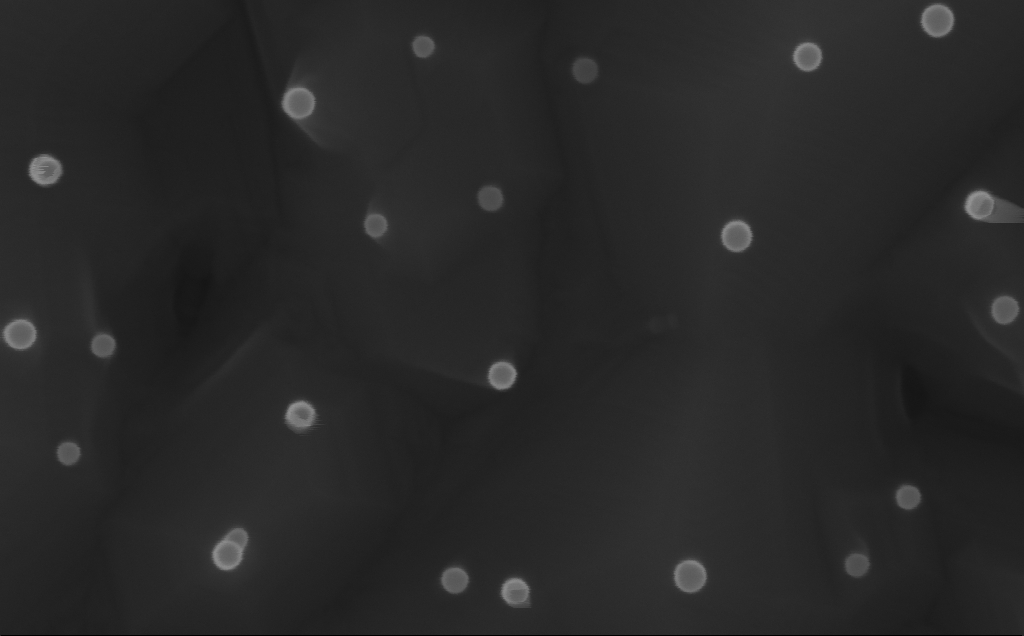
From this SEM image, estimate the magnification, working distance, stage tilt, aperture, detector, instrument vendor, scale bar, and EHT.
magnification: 304.19 K X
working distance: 6 mm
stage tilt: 0°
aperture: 30 µm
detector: InLens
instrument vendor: Zeiss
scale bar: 200 nm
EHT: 10 kV